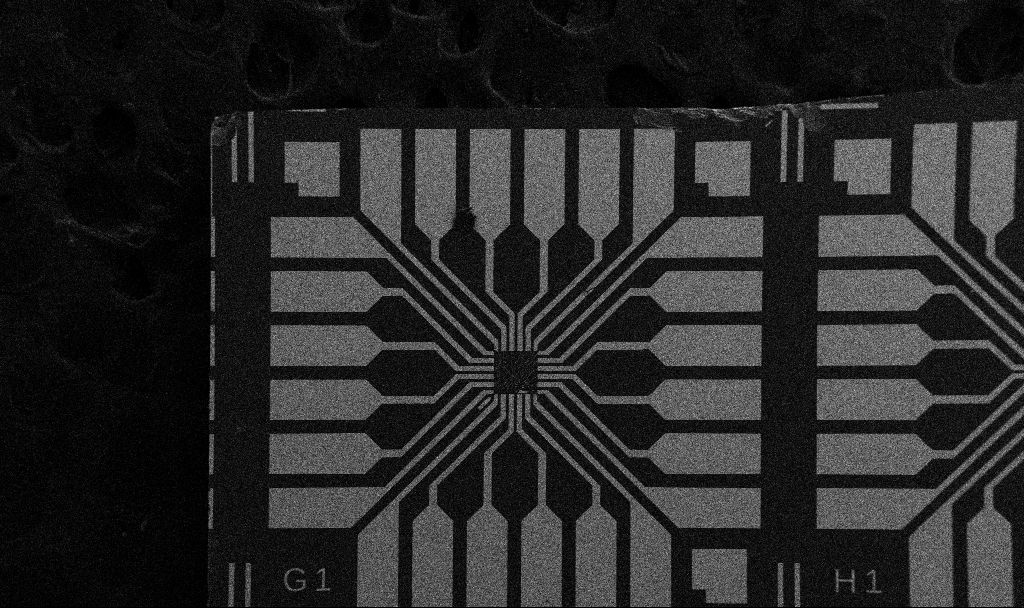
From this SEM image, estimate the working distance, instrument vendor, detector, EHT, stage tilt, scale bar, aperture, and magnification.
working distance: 10.7 mm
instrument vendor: Zeiss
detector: SE2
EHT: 5 kV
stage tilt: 0°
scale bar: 200000 nm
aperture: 30 µm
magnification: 0.1 K X